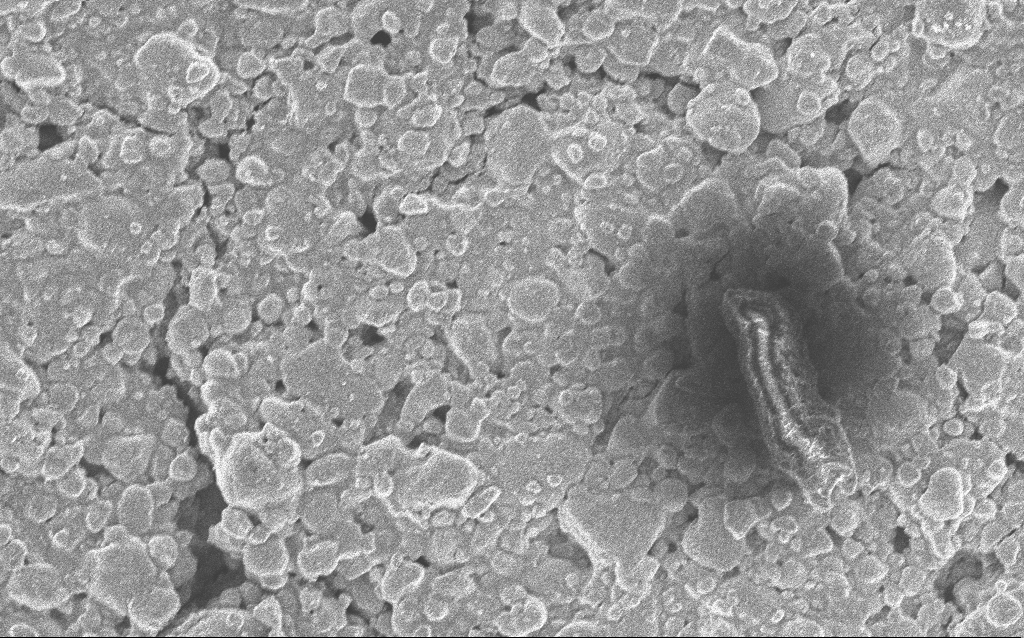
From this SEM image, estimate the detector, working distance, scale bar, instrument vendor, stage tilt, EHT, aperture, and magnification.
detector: InLens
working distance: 4.8 mm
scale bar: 10000 nm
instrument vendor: Zeiss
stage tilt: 0°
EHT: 5 kV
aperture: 30 µm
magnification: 3.88 K X